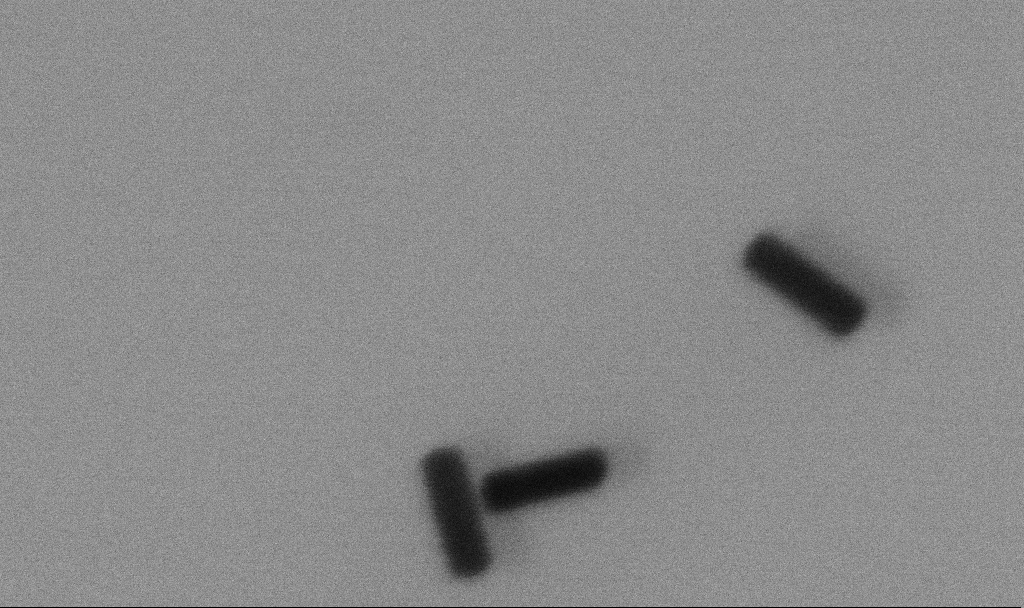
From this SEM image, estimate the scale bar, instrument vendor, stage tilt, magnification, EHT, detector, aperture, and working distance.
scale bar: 100 nm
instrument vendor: Zeiss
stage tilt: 0°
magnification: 632.02 K X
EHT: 5 kV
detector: SE2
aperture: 30 µm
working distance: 4.5 mm